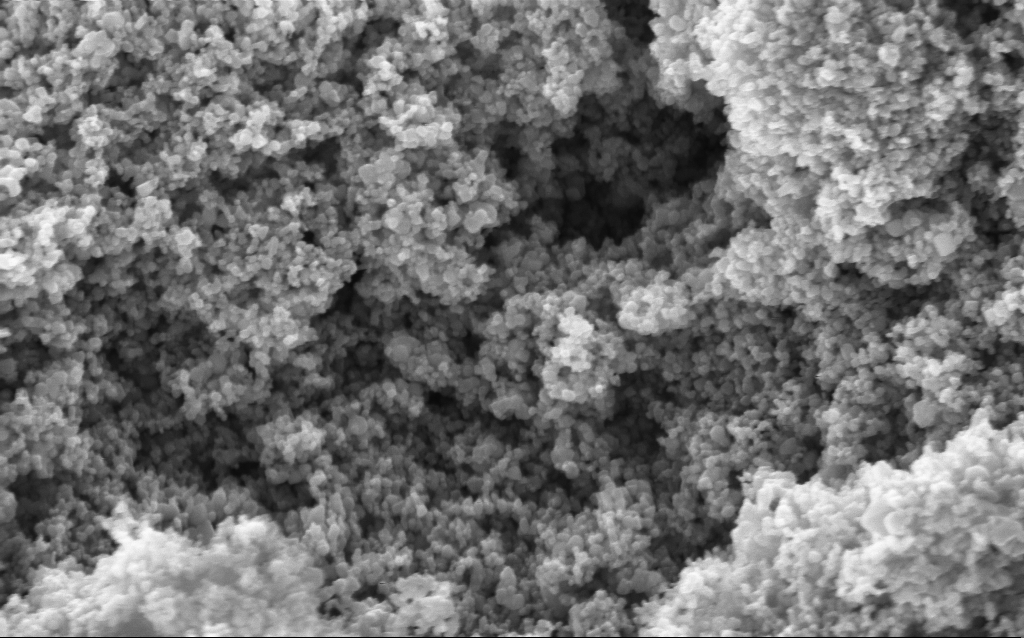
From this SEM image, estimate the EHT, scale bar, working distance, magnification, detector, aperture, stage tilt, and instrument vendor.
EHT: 5 kV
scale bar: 200 nm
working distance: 4.4 mm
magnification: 114.73 K X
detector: InLens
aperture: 30 µm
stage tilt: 0°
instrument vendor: Zeiss